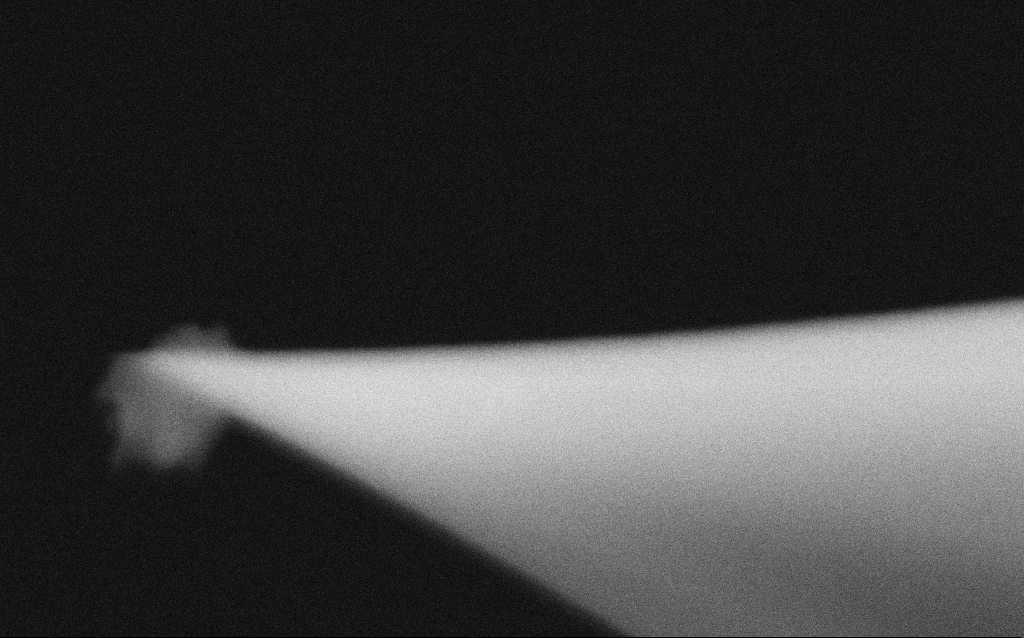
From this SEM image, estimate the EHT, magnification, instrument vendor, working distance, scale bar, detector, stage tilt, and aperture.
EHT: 5 kV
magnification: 250 K X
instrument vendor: Zeiss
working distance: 5.2 mm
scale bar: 100 nm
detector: SE2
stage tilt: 0°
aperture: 30 µm